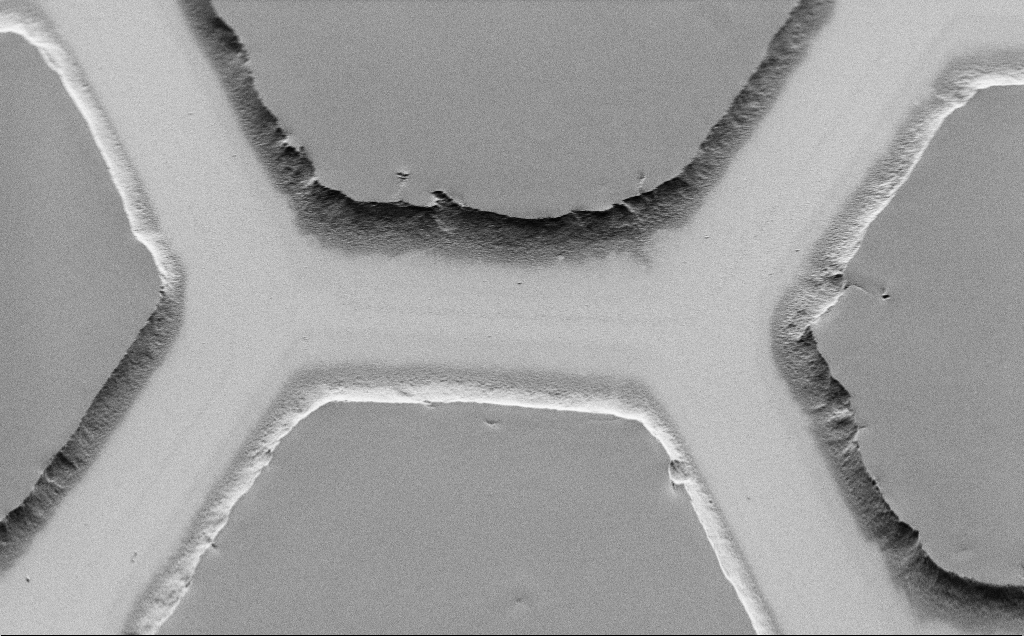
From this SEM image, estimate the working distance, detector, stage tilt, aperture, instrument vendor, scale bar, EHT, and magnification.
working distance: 7 mm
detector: SE2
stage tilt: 45°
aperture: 30 µm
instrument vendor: Zeiss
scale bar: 10000 nm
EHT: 1.5 kV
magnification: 4.42 K X